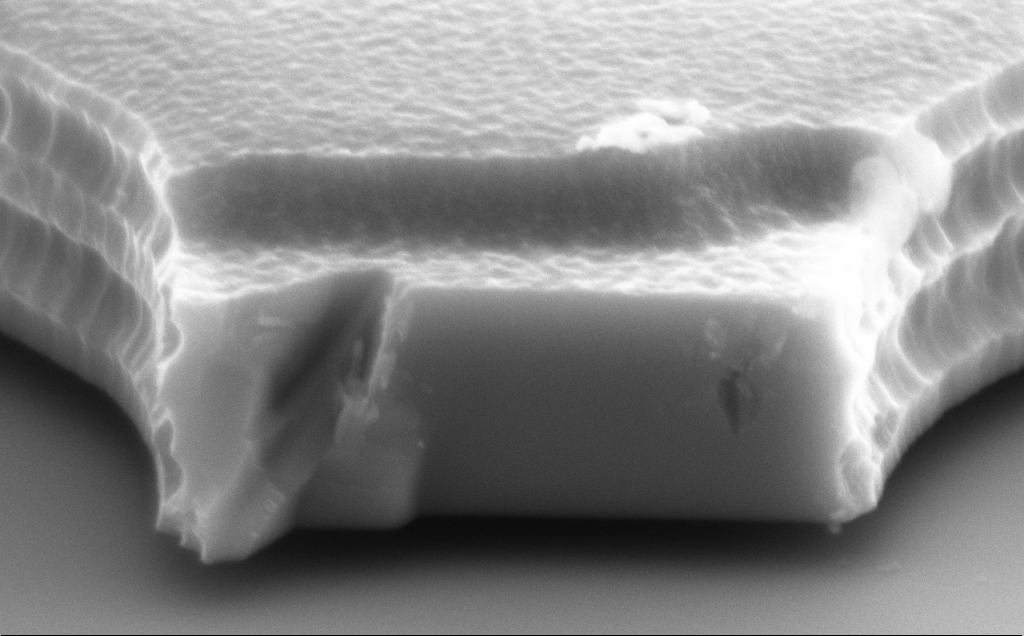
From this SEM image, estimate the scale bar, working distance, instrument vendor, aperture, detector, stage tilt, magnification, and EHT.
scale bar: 1000 nm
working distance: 12 mm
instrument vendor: Zeiss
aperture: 30 µm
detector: SE2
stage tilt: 70°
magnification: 54.58 K X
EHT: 8 kV